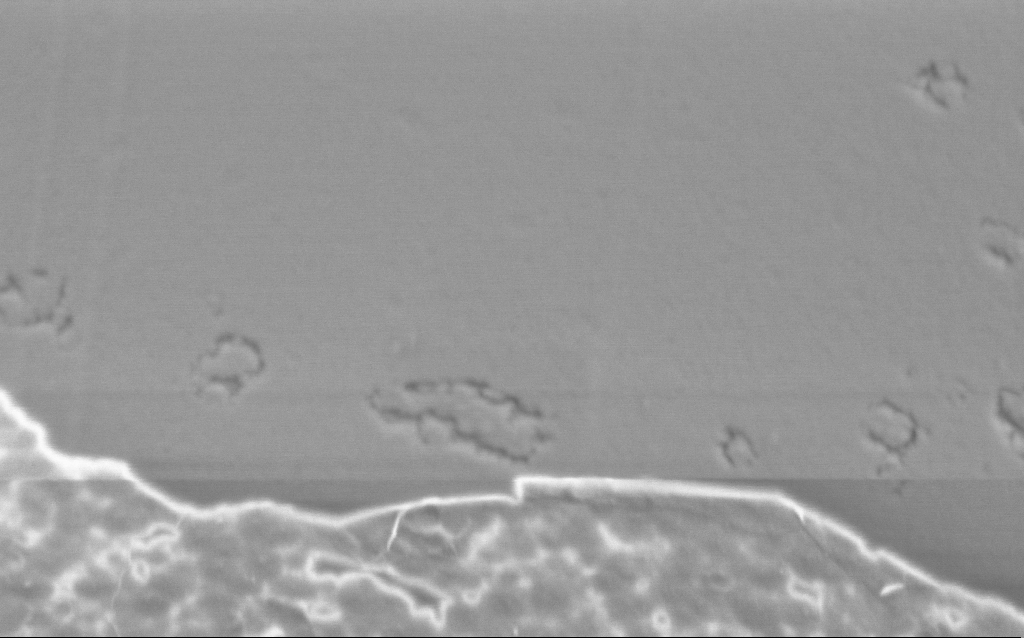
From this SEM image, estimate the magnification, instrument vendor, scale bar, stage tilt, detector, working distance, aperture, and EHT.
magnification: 100 K X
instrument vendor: Zeiss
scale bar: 200 nm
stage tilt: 45°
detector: InLens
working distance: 6 mm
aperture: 30 µm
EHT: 1 kV